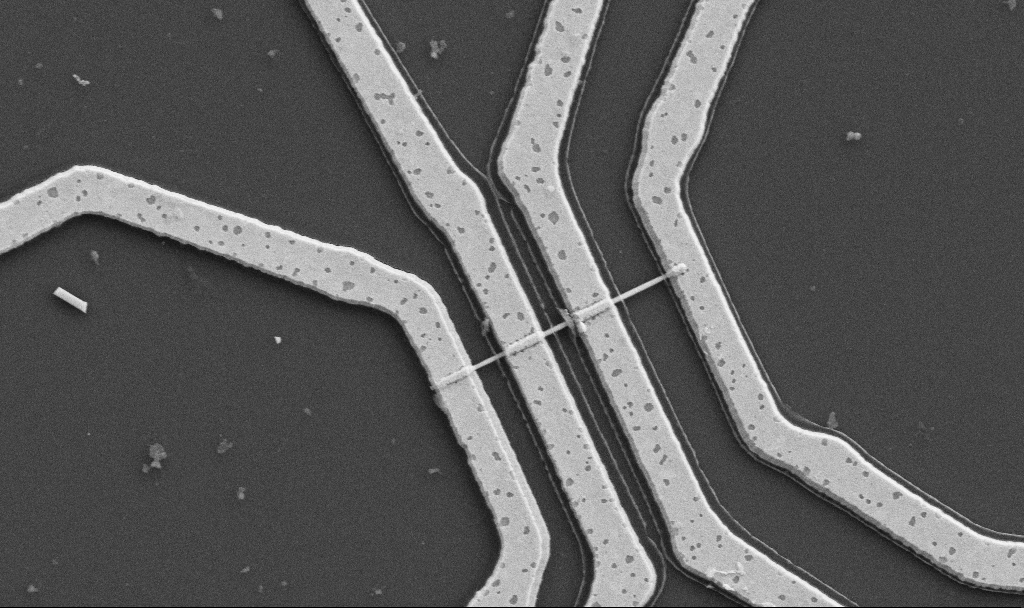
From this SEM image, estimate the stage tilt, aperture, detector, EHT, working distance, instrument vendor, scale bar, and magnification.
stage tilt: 0°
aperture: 30 µm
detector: SE2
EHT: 5 kV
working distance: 10.7 mm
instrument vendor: Zeiss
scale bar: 1000 nm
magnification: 20 K X